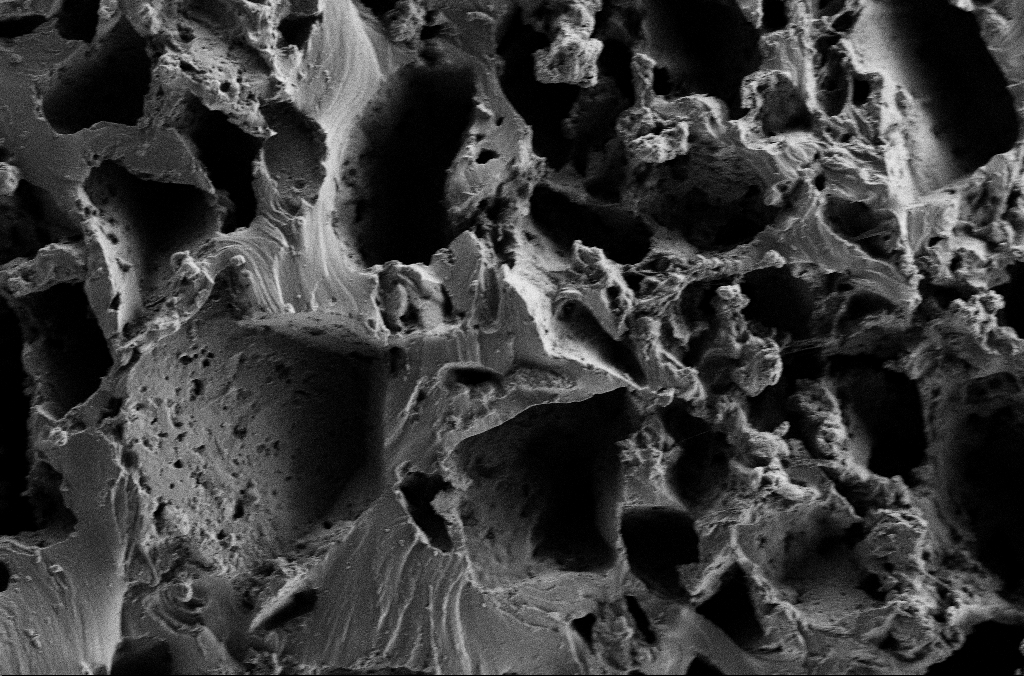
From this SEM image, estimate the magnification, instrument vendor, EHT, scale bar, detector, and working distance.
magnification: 1 K X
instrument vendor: Zeiss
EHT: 2 kV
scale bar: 20000 nm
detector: SE2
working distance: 2.9 mm